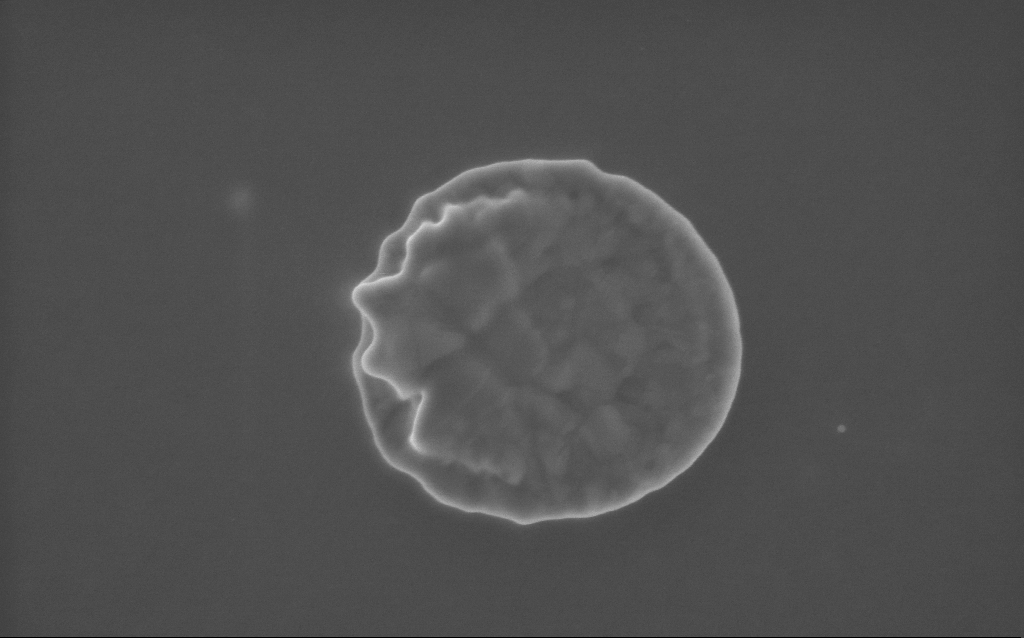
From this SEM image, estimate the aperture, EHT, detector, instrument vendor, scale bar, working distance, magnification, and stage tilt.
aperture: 30 µm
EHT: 5 kV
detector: InLens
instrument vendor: Zeiss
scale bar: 200 nm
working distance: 2 mm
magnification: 77.66 K X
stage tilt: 0°